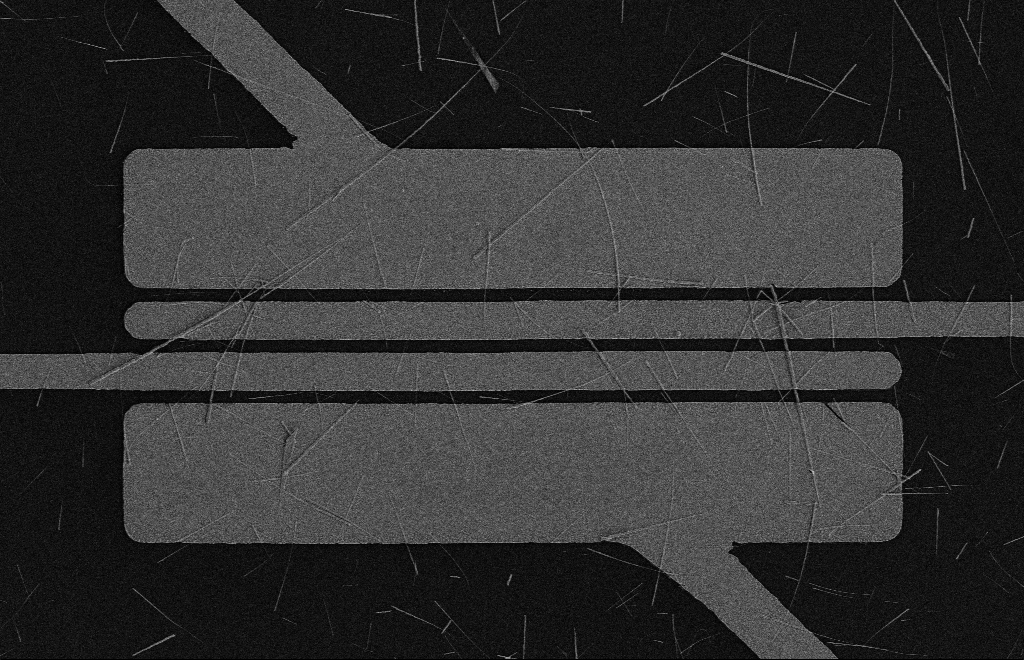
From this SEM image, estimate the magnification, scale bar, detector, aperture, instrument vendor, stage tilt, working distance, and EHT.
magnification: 4.69 K X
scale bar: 10000 nm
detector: SE2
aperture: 10 µm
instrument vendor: Zeiss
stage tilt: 0°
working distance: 16 mm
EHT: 5 kV